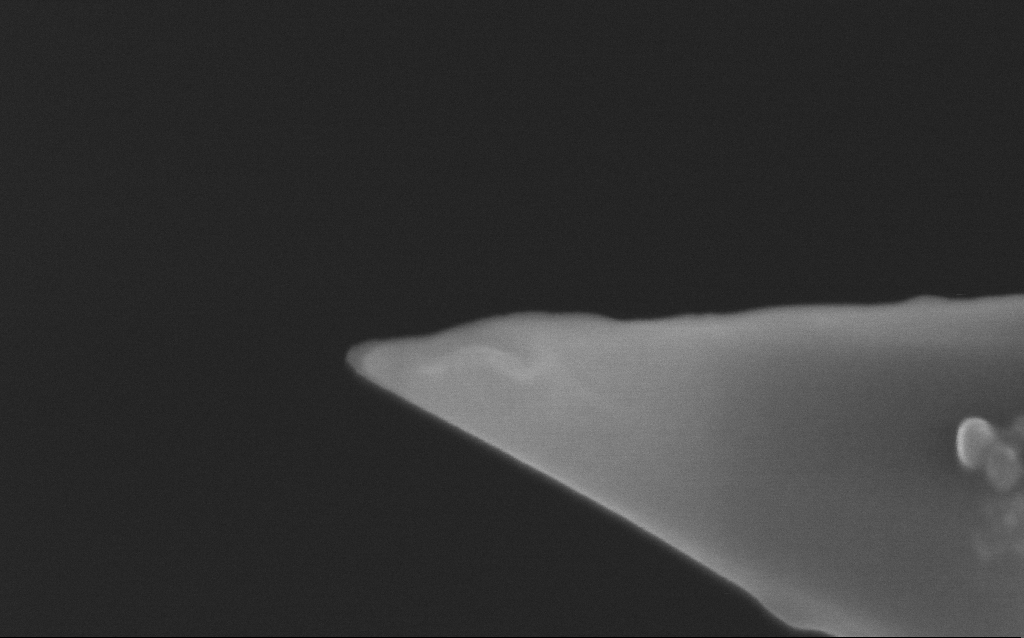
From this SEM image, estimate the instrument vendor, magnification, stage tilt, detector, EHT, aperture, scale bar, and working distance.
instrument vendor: Zeiss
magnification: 250 K X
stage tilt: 0°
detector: InLens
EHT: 5 kV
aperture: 30 µm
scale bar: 100 nm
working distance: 4.7 mm